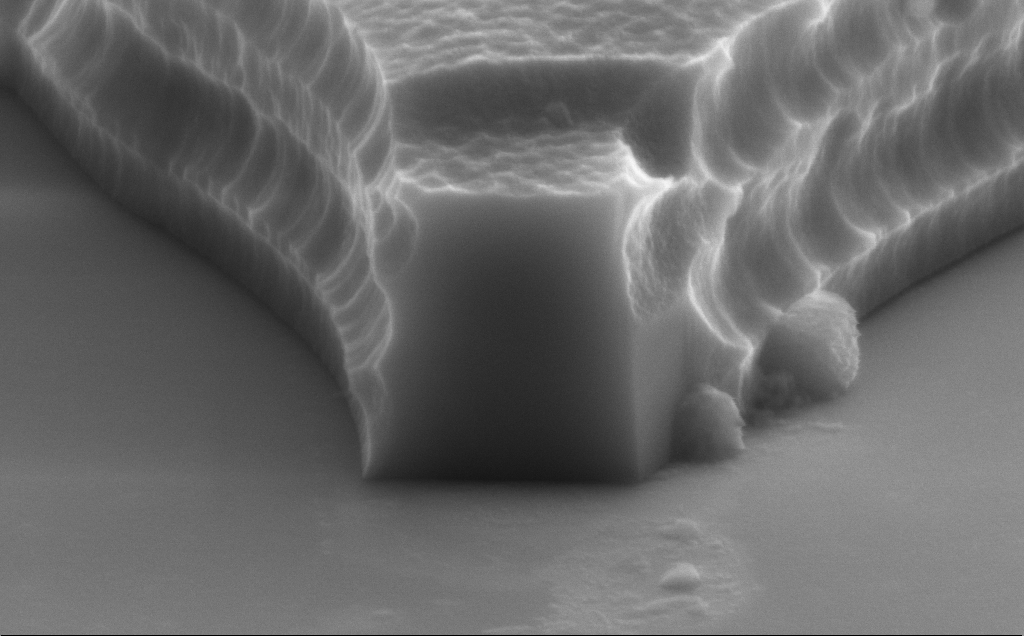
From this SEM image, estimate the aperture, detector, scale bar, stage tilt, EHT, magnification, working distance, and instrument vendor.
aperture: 30 µm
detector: SE2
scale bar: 1000 nm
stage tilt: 70°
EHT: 8 kV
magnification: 65.5 K X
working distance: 12 mm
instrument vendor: Zeiss